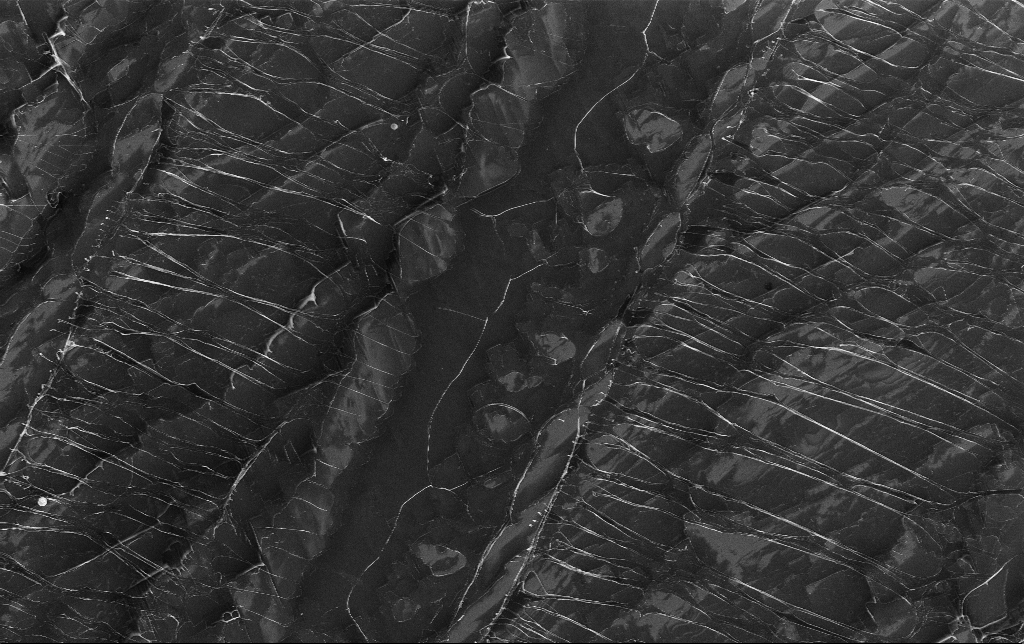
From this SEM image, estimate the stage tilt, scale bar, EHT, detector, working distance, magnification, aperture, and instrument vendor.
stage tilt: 0°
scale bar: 20000 nm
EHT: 5 kV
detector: InLens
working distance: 3.8 mm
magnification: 1.75 K X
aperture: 30 µm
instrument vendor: Zeiss